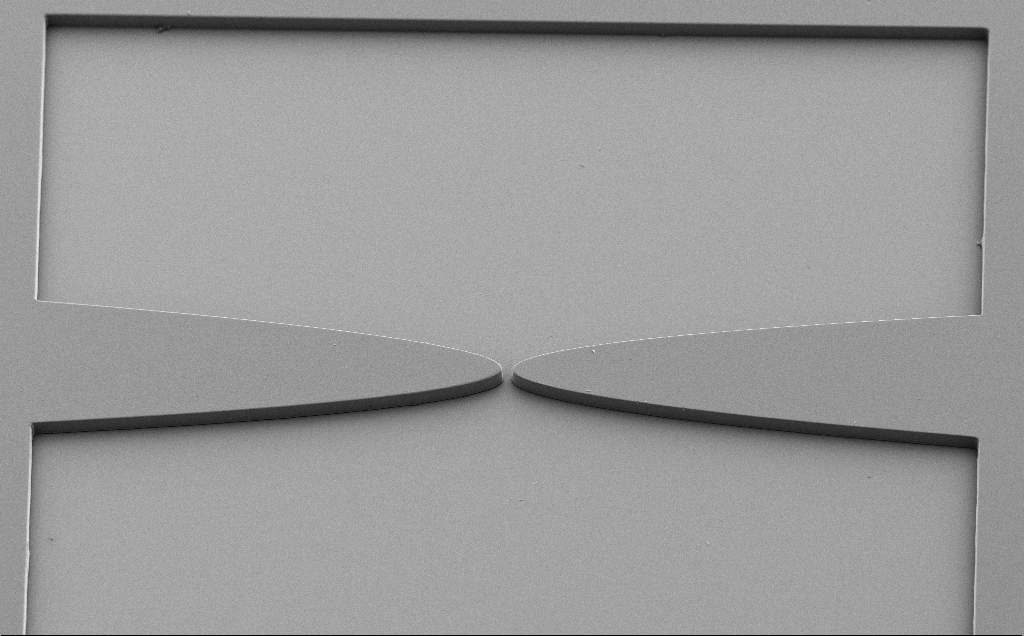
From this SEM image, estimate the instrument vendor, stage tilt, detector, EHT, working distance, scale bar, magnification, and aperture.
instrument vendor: Zeiss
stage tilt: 40°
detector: SE2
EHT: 5 kV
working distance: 8 mm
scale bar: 100000 nm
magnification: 0.64 K X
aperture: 30 µm